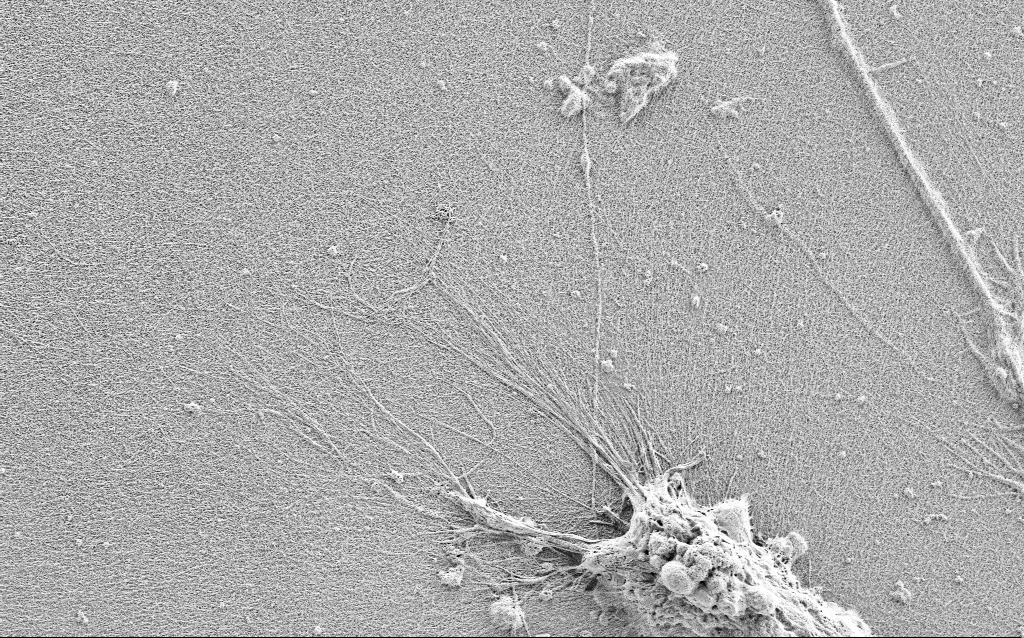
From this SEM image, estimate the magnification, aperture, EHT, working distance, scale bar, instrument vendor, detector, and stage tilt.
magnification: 5 K X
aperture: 30 µm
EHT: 0.9 kV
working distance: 3 mm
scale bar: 10000 nm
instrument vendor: Zeiss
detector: SE2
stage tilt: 0°